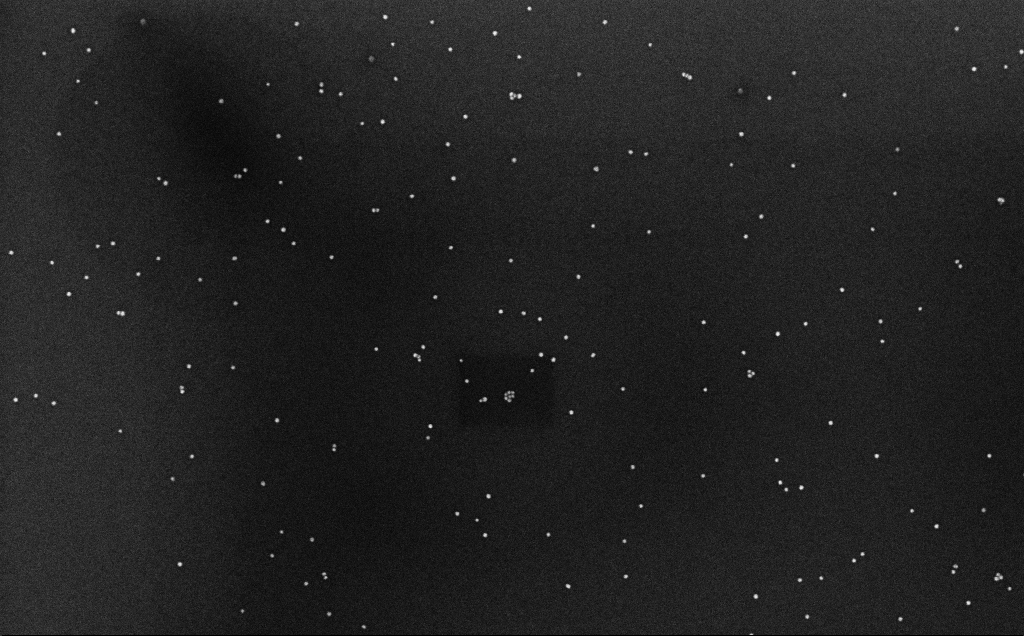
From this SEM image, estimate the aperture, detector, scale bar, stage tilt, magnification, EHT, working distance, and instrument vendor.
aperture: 30 µm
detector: InLens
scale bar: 200 nm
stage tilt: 0°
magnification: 100 K X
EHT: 10 kV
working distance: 6.6 mm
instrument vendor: Zeiss